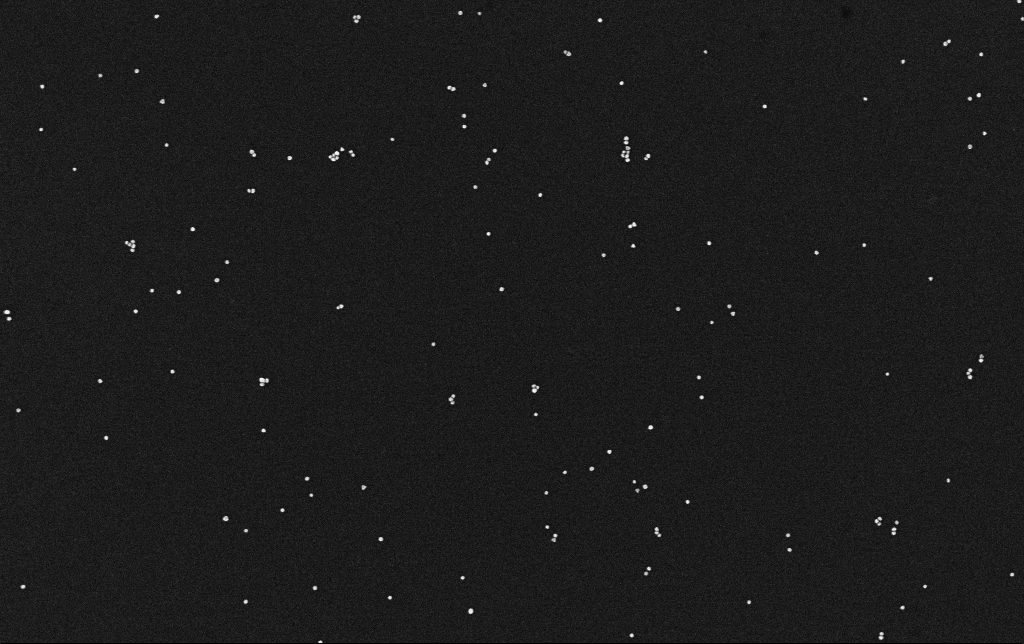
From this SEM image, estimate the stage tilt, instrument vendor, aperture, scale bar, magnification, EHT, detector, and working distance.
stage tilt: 0°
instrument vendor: Zeiss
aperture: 30 µm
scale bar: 200 nm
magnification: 100 K X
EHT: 10 kV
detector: InLens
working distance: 3.1 mm